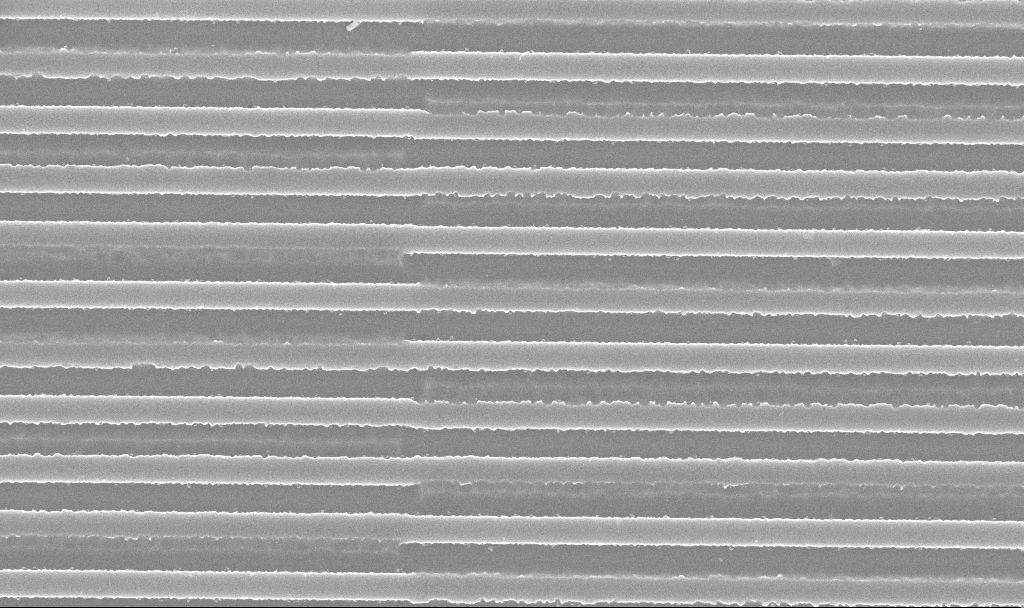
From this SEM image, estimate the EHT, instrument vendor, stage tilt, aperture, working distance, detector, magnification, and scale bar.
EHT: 5 kV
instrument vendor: Zeiss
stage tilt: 0°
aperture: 30 µm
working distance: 9.9 mm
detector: InLens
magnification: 32.43 K X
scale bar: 2000 nm